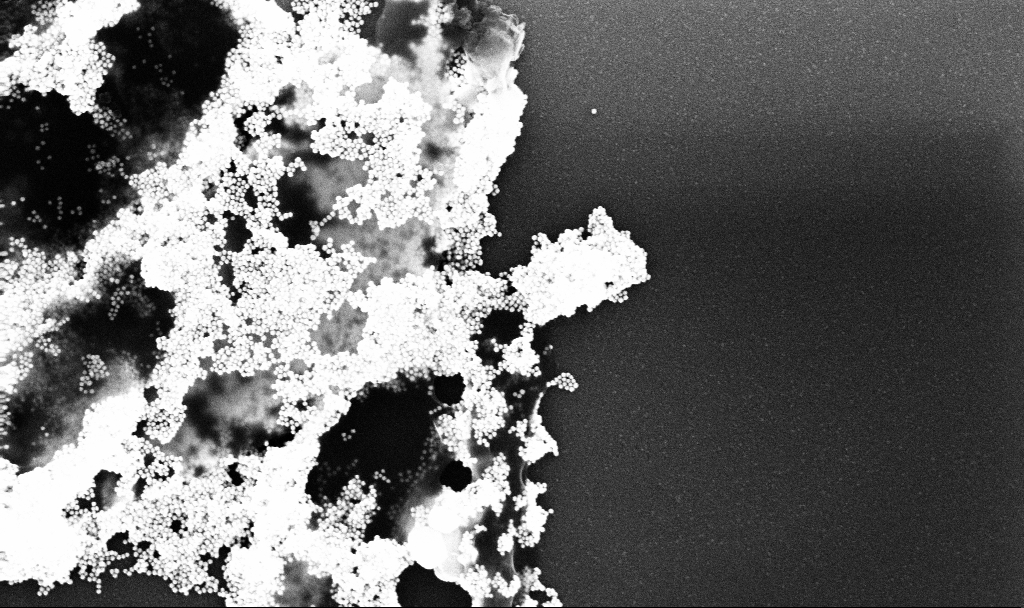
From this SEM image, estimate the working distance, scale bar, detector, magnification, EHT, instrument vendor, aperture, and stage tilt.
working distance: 3.1 mm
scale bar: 200 nm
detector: InLens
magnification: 80 K X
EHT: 10 kV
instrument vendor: Zeiss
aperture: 30 µm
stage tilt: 0°